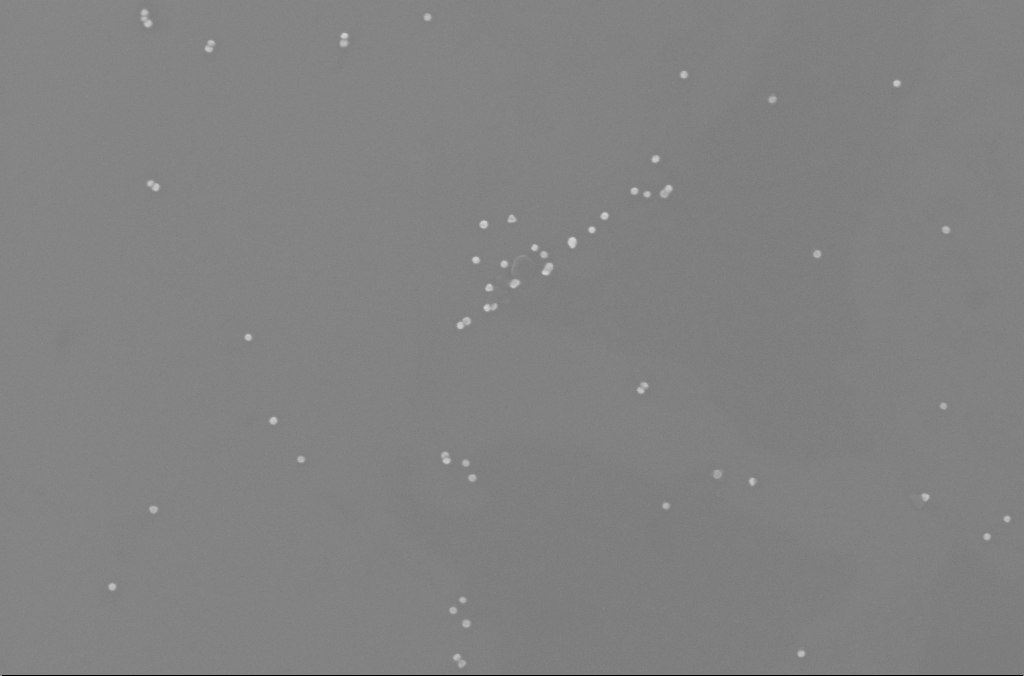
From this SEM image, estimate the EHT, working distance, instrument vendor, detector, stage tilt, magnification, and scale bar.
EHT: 10 kV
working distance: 2.5 mm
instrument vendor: Zeiss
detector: InLens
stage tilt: -0°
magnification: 128.44 K X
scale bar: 200 nm